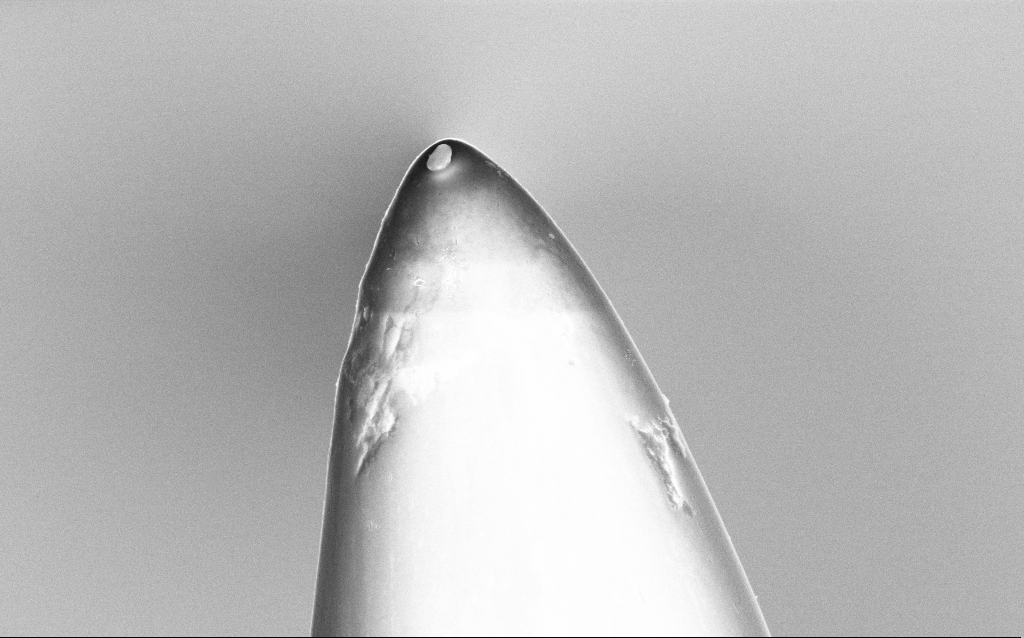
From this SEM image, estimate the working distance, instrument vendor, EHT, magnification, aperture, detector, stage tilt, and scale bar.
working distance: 2.9 mm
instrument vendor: Zeiss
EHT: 5 kV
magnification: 32.88 K X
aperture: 30 µm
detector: InLens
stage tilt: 0°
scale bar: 1000 nm